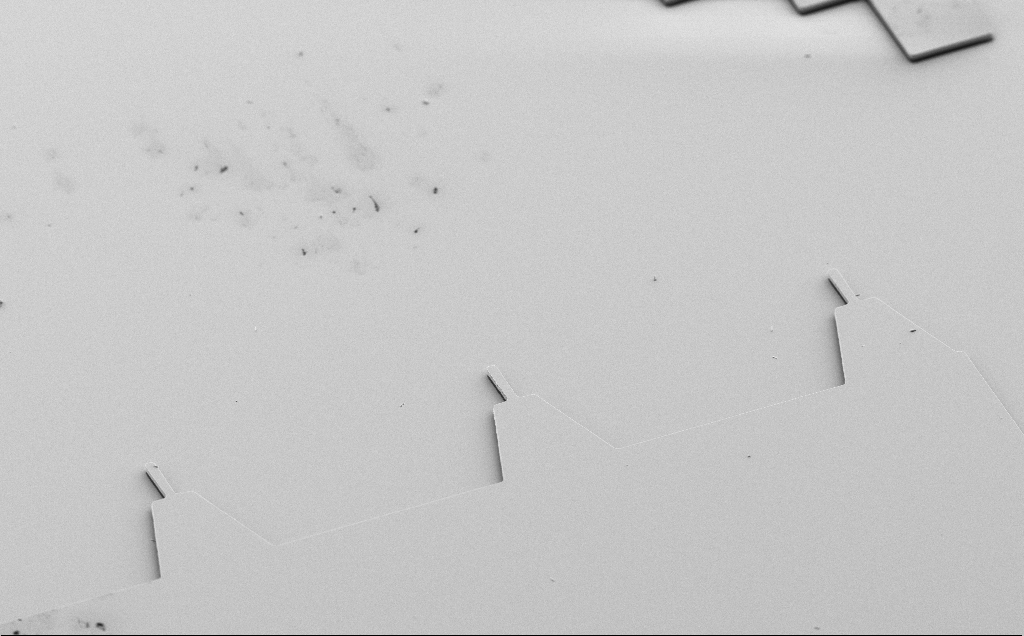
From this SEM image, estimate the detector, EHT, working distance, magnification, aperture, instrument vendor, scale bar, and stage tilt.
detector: SE2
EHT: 5 kV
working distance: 10 mm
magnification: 0.855 K X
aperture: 30 µm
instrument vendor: Zeiss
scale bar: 20000 nm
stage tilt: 50°